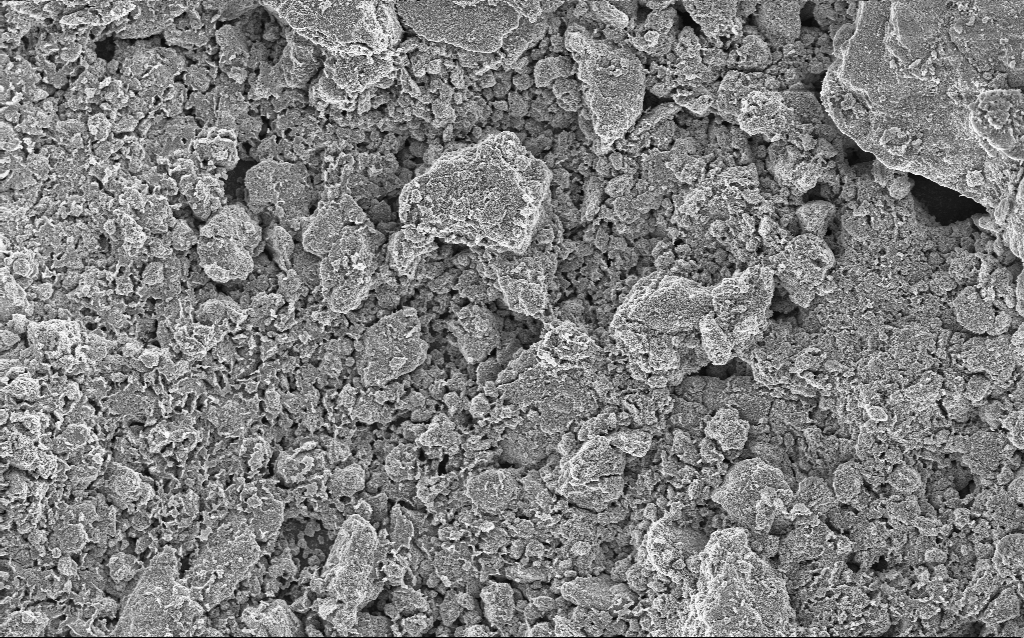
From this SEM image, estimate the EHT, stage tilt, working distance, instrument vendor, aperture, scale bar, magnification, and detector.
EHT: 5 kV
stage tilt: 0°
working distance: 4.4 mm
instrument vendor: Zeiss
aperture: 30 µm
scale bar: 20000 nm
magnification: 1.23 K X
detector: InLens